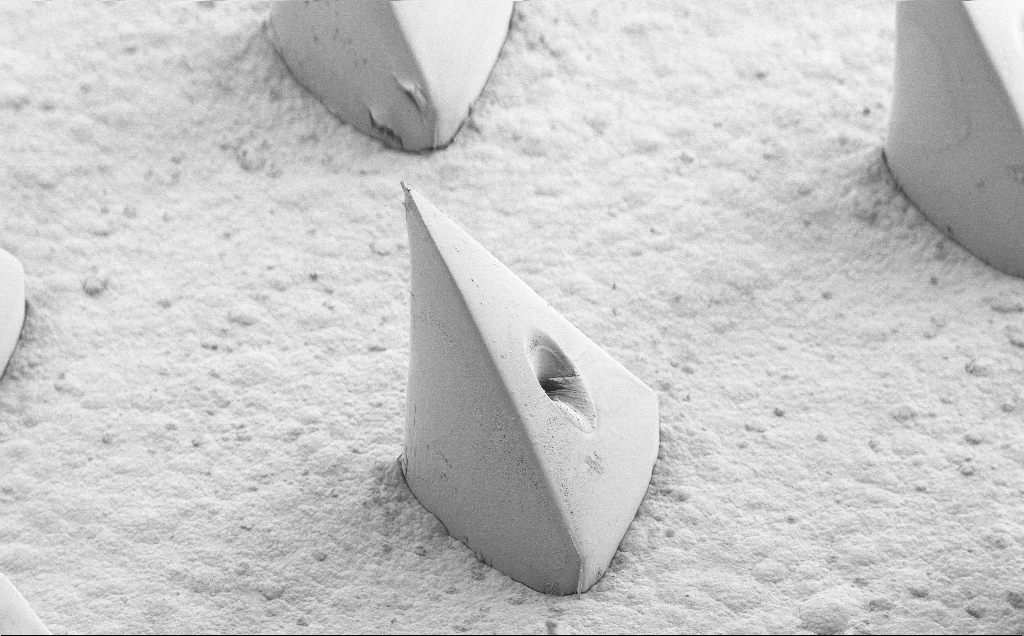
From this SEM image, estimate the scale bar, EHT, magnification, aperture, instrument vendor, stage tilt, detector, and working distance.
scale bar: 100000 nm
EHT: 5 kV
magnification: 0.165 K X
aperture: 30 µm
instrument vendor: Zeiss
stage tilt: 40°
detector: SE2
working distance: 8 mm